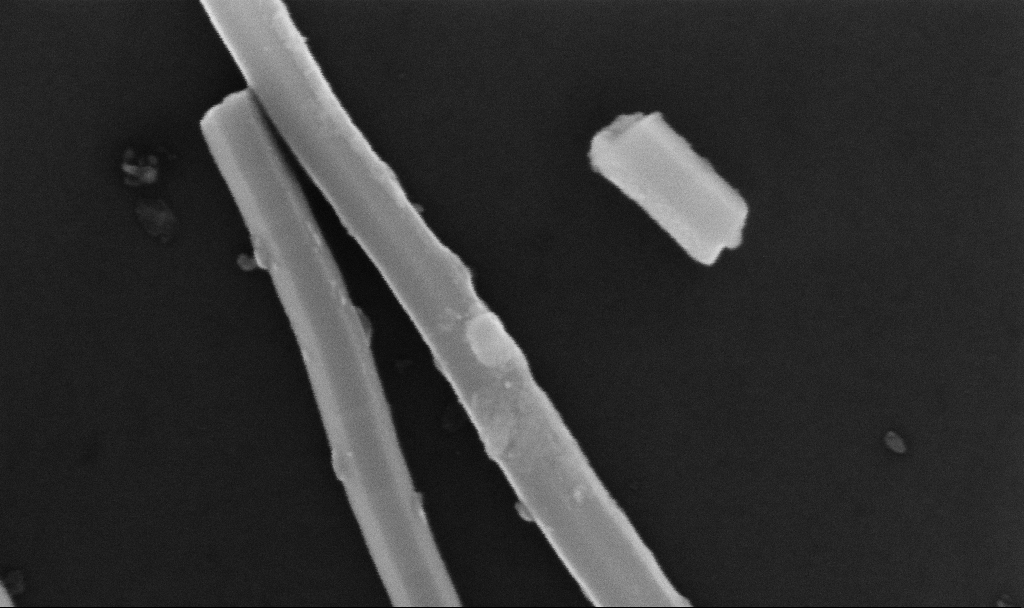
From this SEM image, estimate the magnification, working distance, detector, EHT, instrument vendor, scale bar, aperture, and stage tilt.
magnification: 233.75 K X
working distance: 6.7 mm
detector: InLens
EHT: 10 kV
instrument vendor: Zeiss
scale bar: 200 nm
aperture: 30 µm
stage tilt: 0°